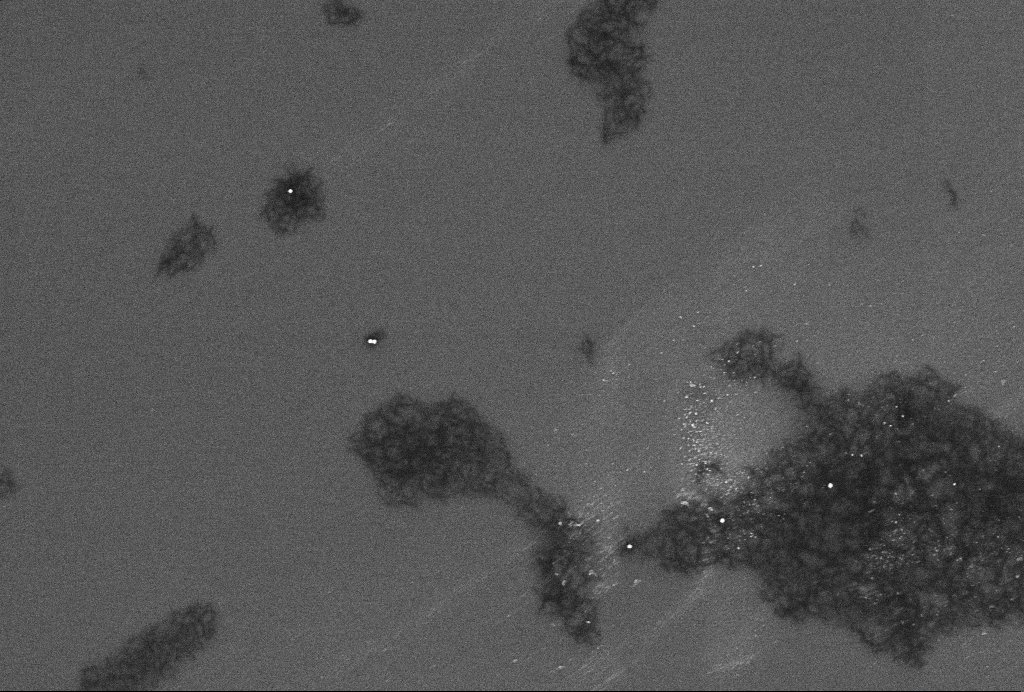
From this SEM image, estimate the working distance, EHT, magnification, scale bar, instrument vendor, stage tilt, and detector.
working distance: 3.3 mm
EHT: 2 kV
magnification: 60.8 K X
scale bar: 200 nm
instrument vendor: Zeiss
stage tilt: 0°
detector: InLens